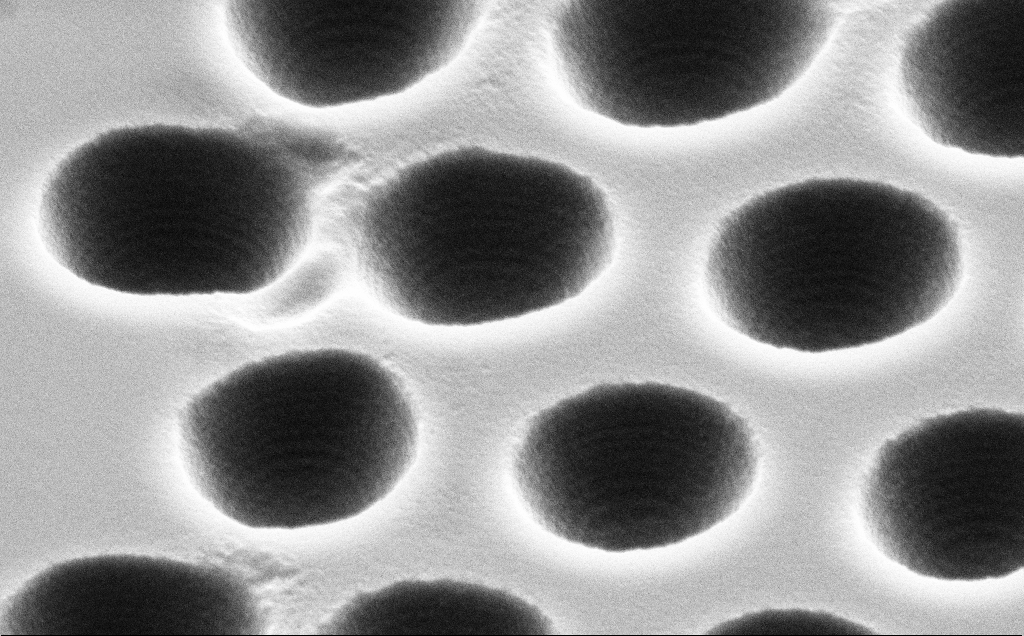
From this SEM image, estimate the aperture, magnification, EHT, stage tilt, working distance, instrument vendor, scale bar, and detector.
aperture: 30 µm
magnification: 39.94 K X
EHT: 5 kV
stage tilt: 45°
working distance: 11 mm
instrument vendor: Zeiss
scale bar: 1000 nm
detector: SE2